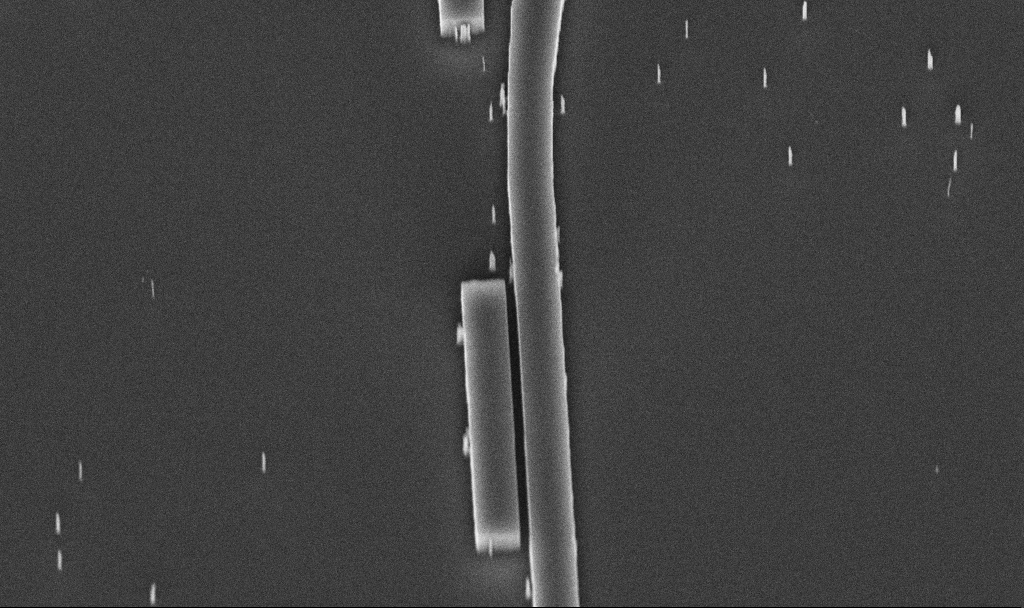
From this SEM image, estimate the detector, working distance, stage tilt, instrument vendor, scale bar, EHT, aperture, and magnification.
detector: InLens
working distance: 9.8 mm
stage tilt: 45°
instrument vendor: Zeiss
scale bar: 2000 nm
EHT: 5 kV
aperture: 30 µm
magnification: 32.43 K X